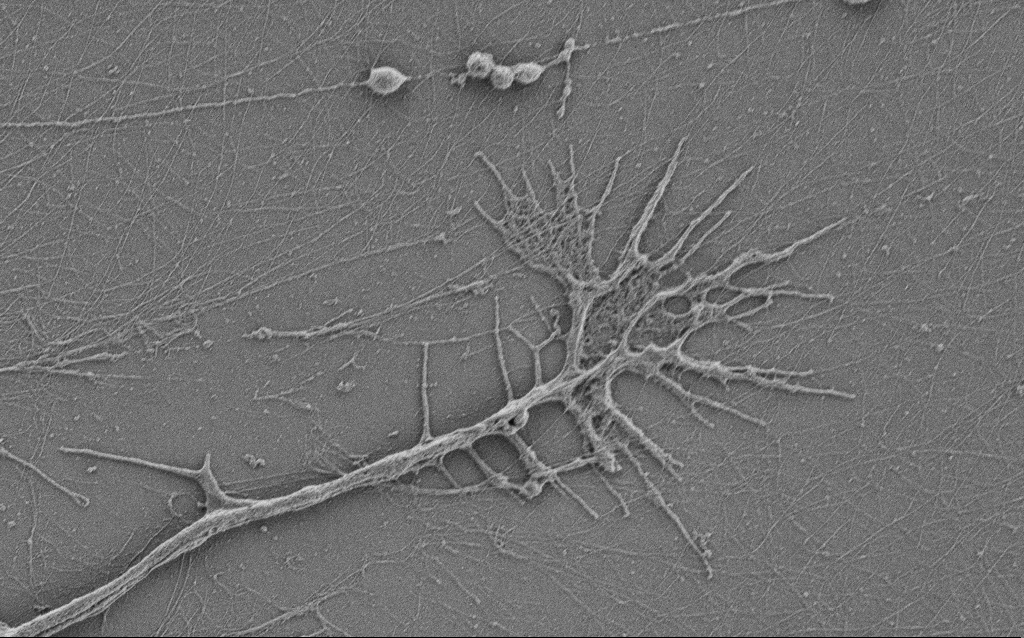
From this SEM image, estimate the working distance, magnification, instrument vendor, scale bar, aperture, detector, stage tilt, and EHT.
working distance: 4 mm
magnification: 10 K X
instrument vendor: Zeiss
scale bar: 2000 nm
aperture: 30 µm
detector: SE2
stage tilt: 0°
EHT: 0.9 kV